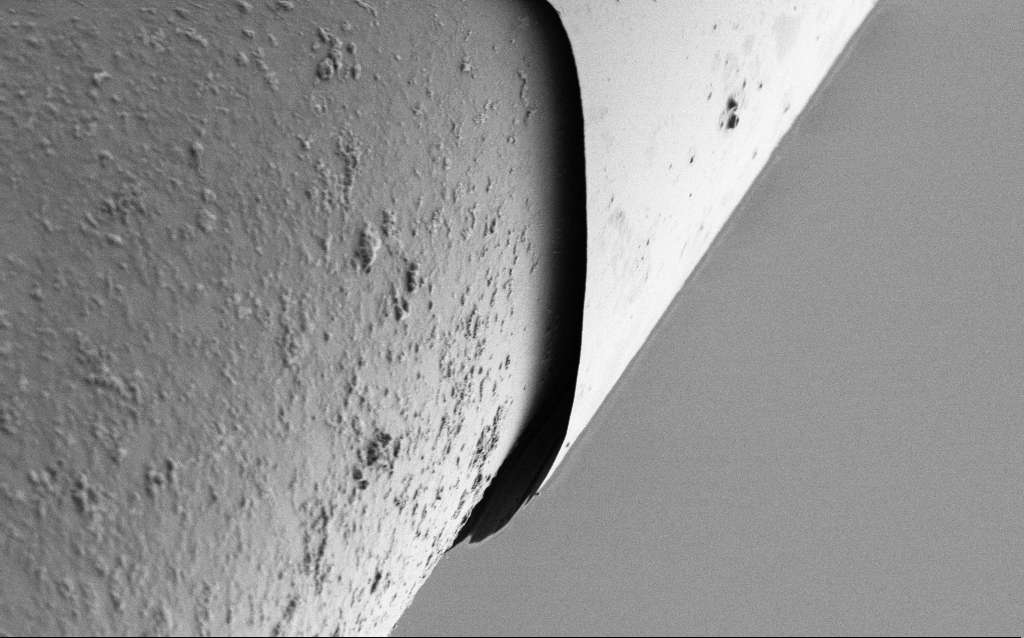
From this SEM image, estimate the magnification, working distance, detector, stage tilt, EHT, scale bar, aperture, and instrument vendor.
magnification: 10 K X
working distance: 7.7 mm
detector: SE2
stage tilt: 45°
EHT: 1 kV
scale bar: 2000 nm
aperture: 30 µm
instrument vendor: Zeiss